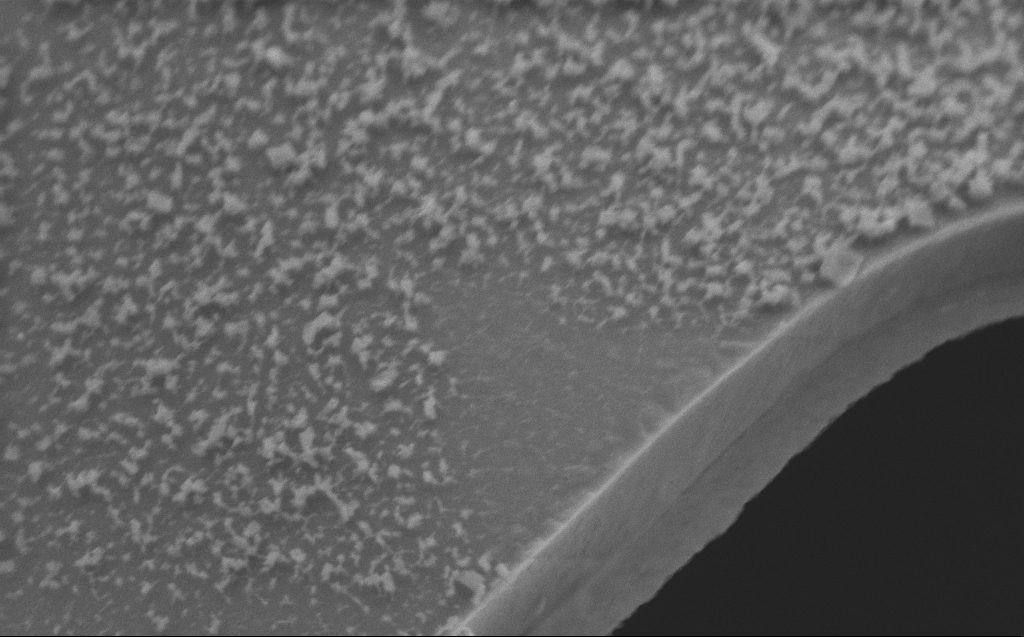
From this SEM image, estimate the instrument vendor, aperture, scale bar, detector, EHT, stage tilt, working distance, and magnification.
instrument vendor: Zeiss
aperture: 30 µm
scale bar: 2000 nm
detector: SE2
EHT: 5 kV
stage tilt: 45°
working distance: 4 mm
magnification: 26.84 K X